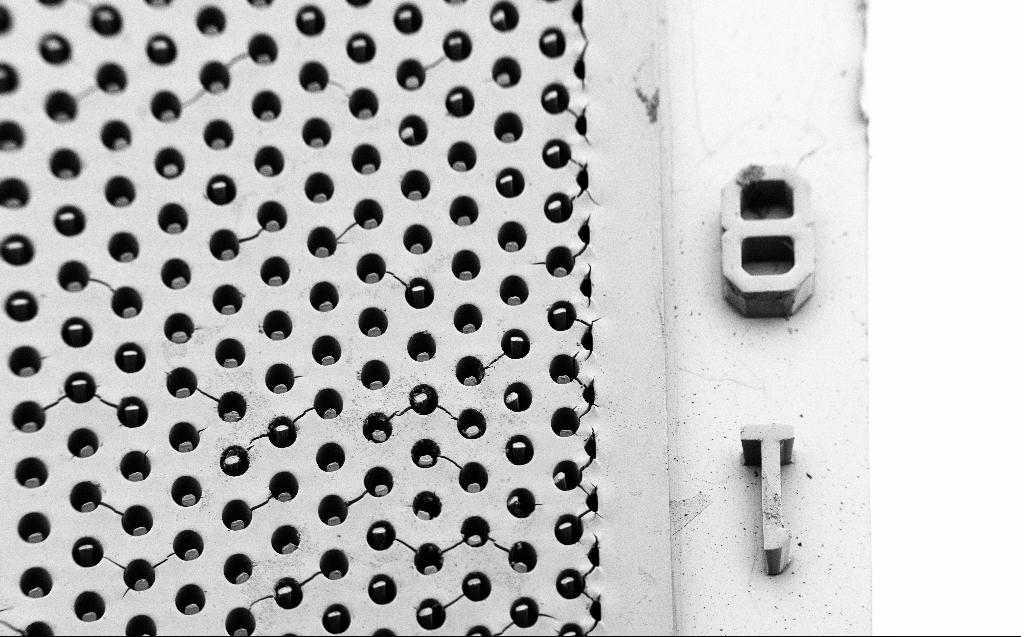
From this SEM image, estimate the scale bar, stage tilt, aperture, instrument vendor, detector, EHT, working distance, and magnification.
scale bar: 100000 nm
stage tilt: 45°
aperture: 30 µm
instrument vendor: Zeiss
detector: SE2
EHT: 1.7 kV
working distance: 5 mm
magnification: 0.188 K X